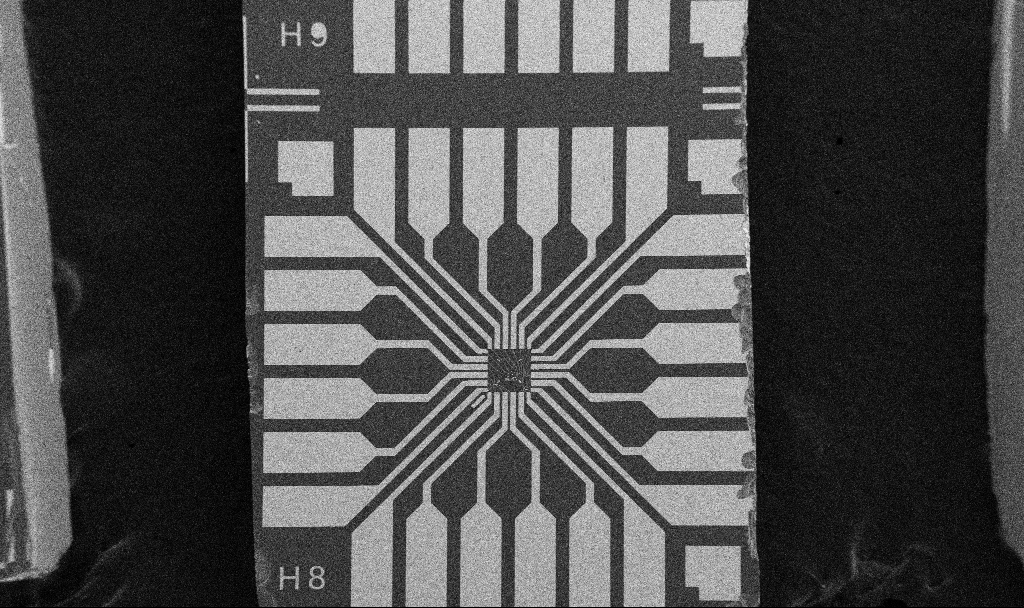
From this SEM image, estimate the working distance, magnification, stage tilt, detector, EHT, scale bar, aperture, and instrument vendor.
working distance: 10.7 mm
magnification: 0.1 K X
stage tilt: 0°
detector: SE2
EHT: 5 kV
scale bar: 200000 nm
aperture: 30 µm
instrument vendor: Zeiss